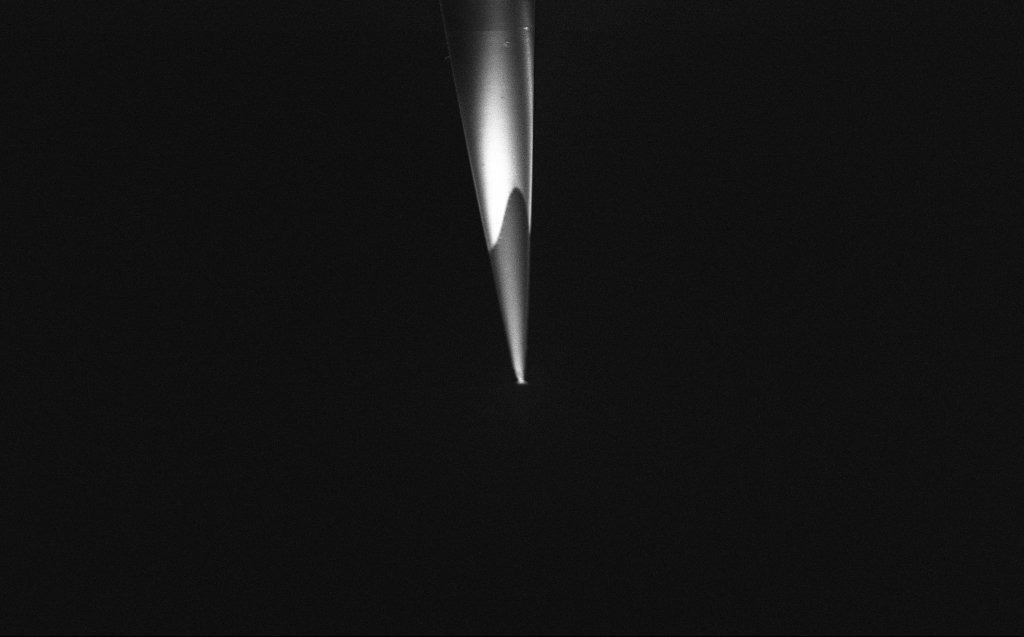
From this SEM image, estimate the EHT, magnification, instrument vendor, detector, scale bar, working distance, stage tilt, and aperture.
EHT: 2 kV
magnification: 10 K X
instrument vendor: Zeiss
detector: InLens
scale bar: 2000 nm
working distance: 6 mm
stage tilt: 45°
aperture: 30 µm